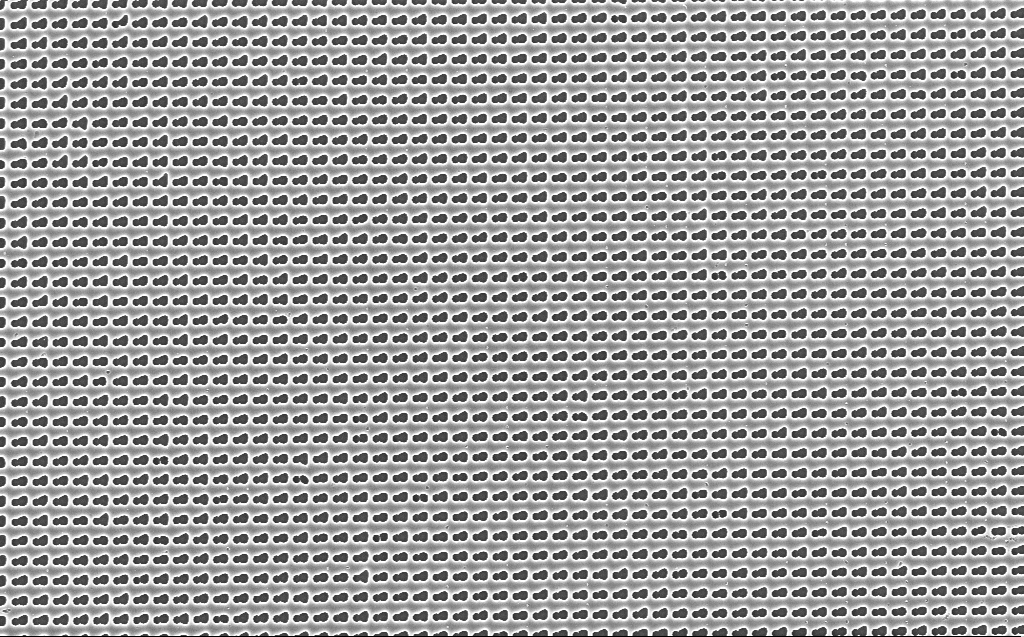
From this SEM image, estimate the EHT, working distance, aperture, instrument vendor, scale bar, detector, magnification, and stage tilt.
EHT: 5 kV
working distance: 4 mm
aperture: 30 µm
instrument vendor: Zeiss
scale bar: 10000 nm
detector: InLens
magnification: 7.05 K X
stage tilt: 0°